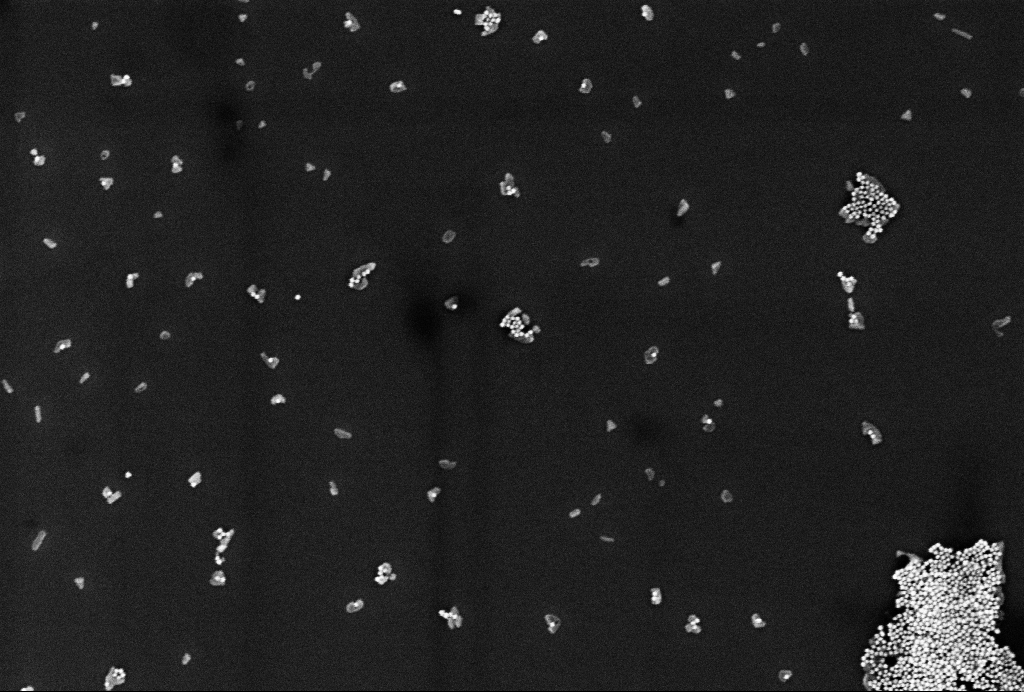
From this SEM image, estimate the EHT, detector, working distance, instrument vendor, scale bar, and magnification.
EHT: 2 kV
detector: InLens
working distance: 3.3 mm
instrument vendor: Zeiss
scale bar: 200 nm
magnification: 79.3 K X